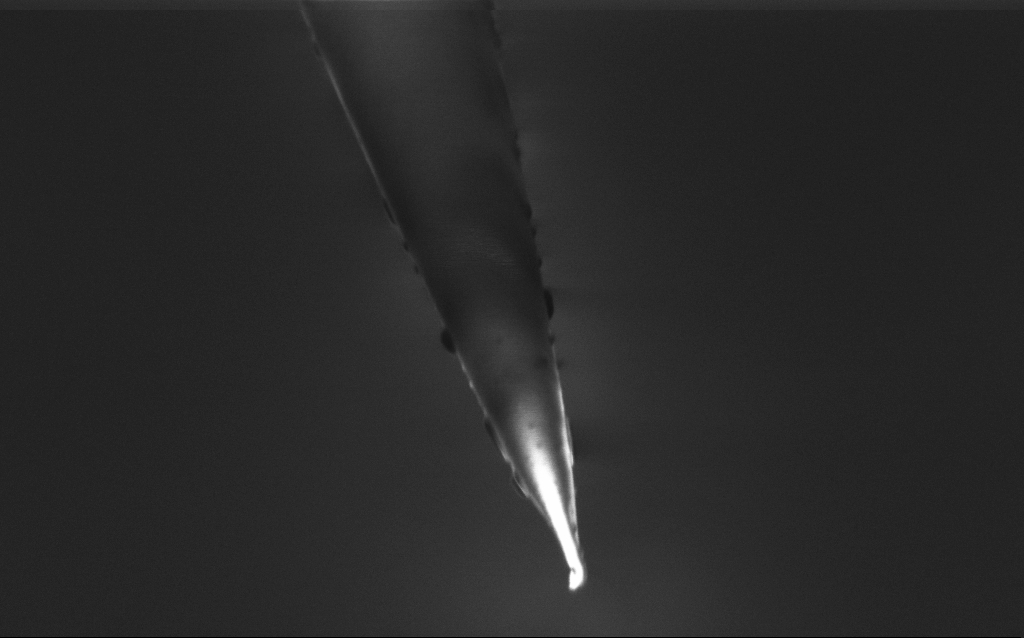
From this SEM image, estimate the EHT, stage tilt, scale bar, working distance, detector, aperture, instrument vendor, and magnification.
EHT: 1 kV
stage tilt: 45°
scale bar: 1000 nm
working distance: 6 mm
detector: InLens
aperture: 30 µm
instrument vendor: Zeiss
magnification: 50 K X